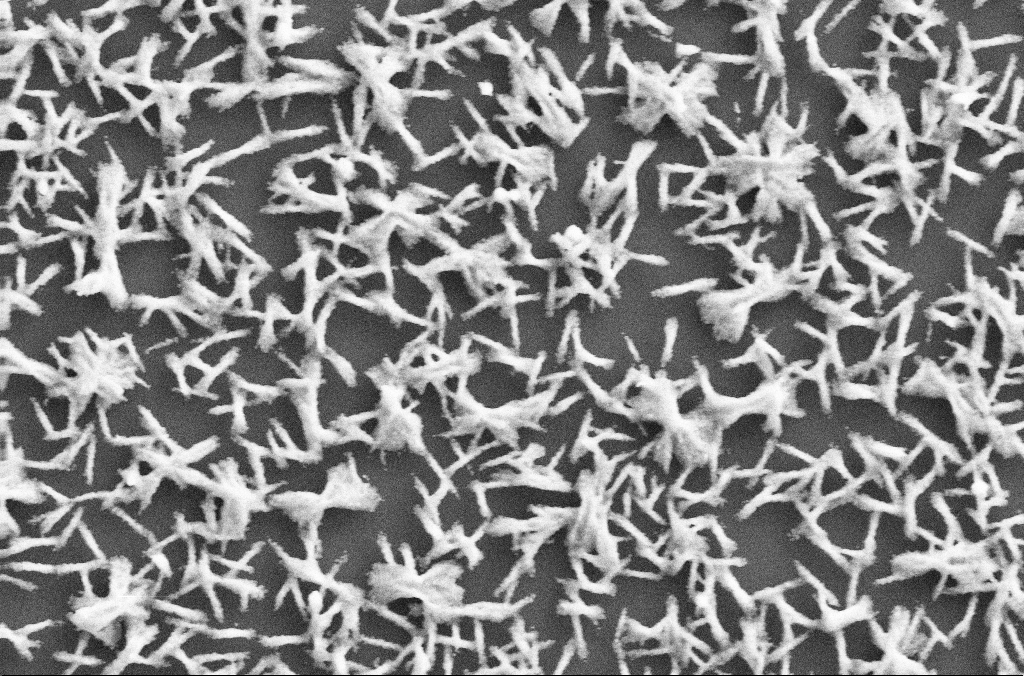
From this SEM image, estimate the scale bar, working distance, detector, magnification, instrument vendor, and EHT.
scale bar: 10000 nm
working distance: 2.8 mm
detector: SE2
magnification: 5 K X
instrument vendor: Zeiss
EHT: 20 kV